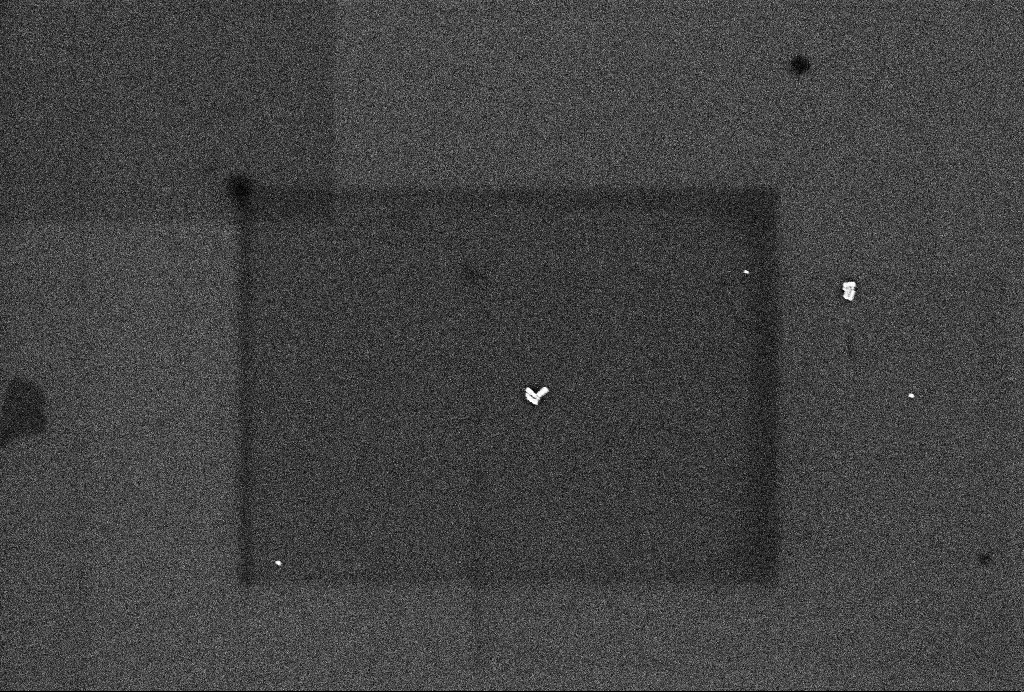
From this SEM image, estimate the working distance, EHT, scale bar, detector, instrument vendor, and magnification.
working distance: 3.3 mm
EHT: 2 kV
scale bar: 200 nm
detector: InLens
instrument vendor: Zeiss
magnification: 60.84 K X